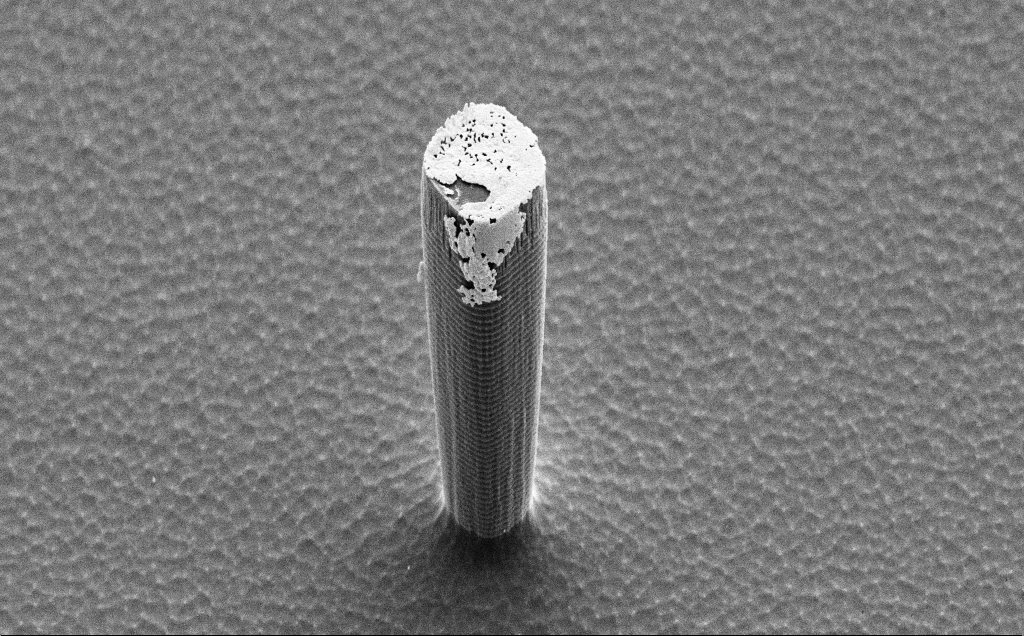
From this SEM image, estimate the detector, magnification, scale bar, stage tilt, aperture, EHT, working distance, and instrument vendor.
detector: SE2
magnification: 6.45 K X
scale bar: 10000 nm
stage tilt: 50°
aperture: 30 µm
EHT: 5 kV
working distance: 10 mm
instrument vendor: Zeiss